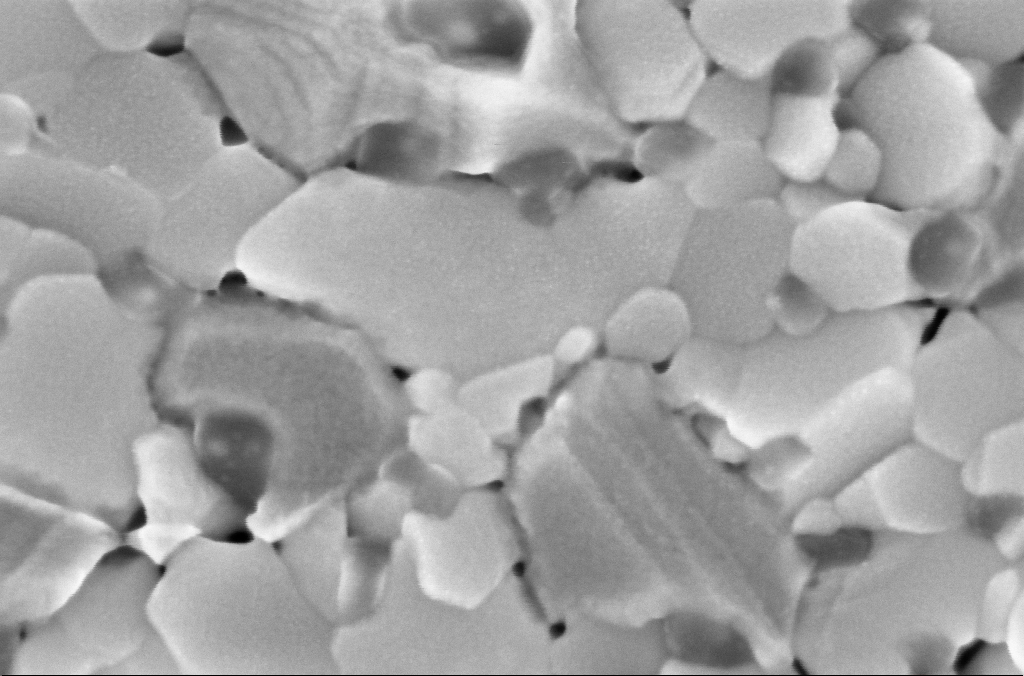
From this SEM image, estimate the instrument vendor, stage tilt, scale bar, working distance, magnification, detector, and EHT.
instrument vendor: Zeiss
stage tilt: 0°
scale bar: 200 nm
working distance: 3 mm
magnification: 267.59 K X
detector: InLens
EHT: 5 kV